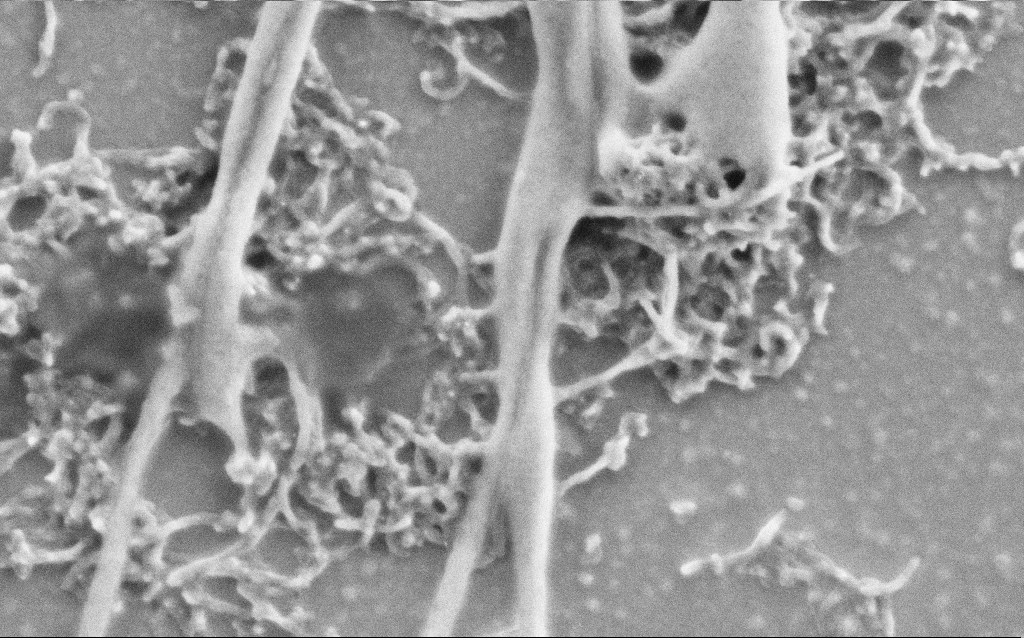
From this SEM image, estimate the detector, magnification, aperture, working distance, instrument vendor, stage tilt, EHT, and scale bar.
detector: SE2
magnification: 75 K X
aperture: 30 µm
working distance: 6.9 mm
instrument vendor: Zeiss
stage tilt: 0°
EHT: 2 kV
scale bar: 200 nm